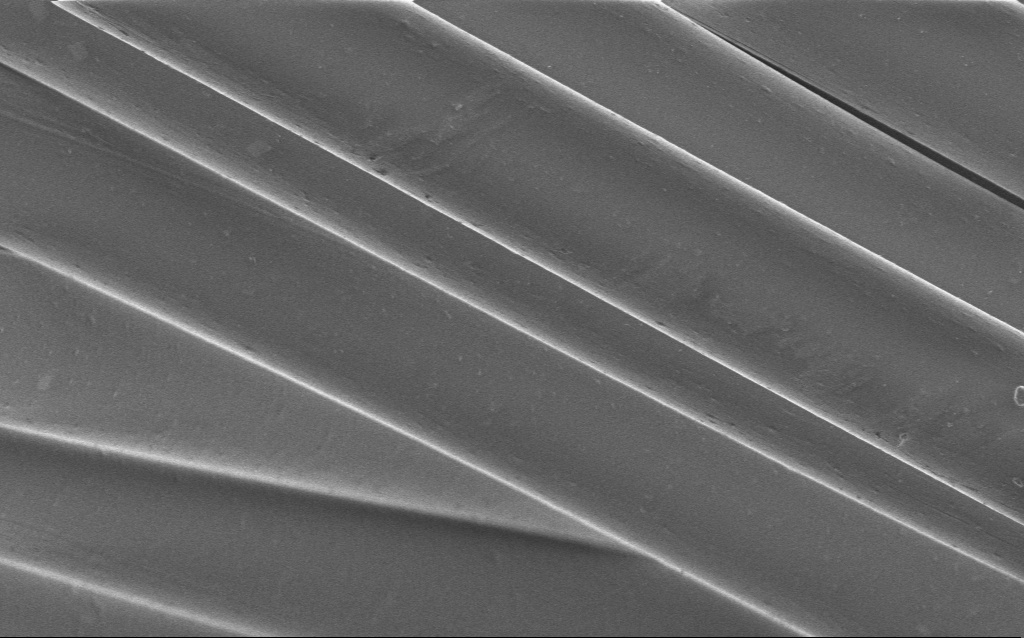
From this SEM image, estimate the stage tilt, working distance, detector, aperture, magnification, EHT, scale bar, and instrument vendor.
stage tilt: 0°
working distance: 4 mm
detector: InLens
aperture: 30 µm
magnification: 2.63 K X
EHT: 1 kV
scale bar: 20000 nm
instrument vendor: Zeiss